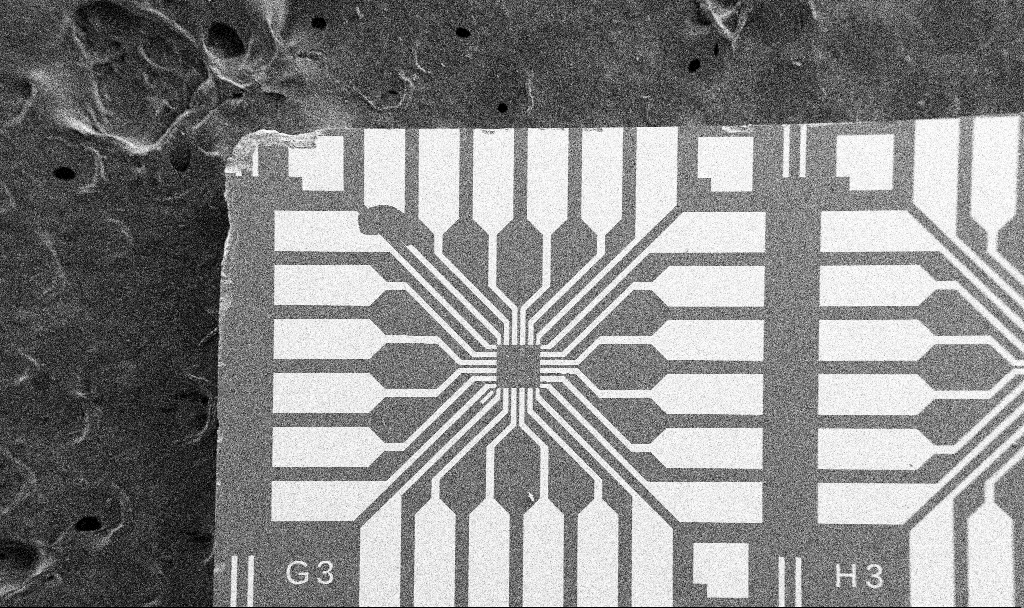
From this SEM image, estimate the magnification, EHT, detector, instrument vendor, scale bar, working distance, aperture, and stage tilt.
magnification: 0.1 K X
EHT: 5 kV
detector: SE2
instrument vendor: Zeiss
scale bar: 200000 nm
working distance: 10.7 mm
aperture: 30 µm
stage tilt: -0°